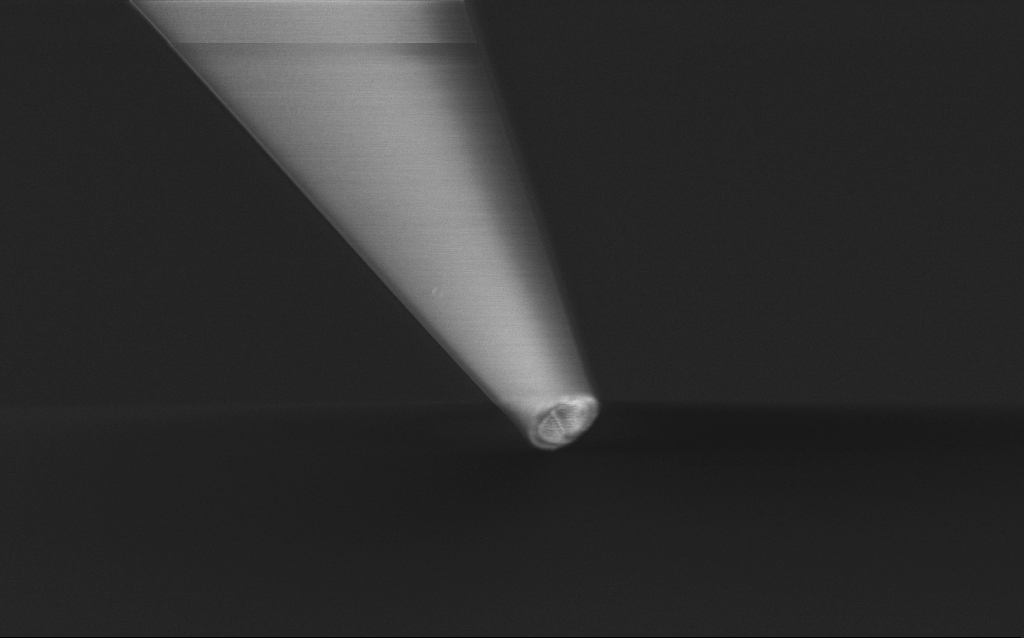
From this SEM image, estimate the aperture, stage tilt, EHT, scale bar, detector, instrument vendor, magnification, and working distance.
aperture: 30 µm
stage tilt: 45°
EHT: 1 kV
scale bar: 2000 nm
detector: InLens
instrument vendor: Zeiss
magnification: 10 K X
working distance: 7 mm